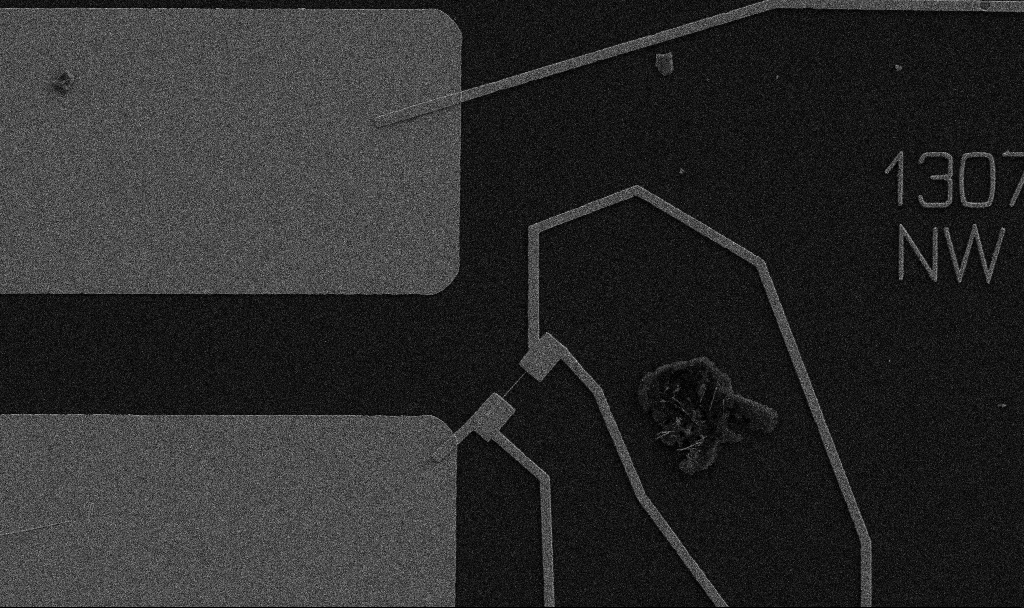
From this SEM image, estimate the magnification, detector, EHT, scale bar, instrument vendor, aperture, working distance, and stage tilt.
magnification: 5 K X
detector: SE2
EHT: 5 kV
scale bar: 10000 nm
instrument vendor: Zeiss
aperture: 30 µm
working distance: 10.7 mm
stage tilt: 0°